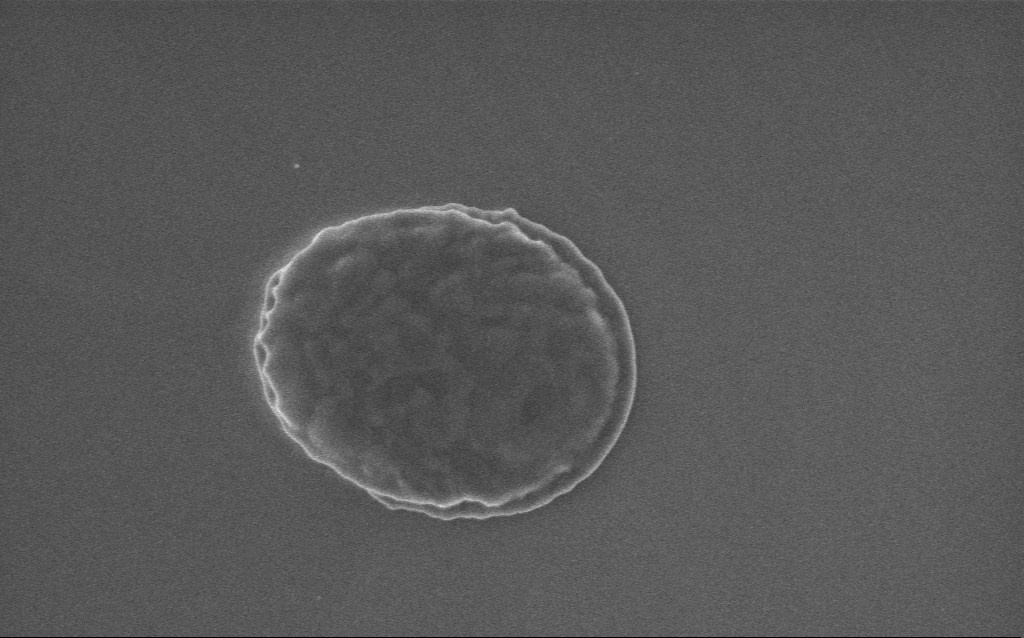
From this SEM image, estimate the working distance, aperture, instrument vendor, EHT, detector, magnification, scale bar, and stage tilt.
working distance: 3 mm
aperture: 30 µm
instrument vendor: Zeiss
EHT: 5 kV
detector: InLens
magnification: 43 K X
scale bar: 1000 nm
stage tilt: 0°